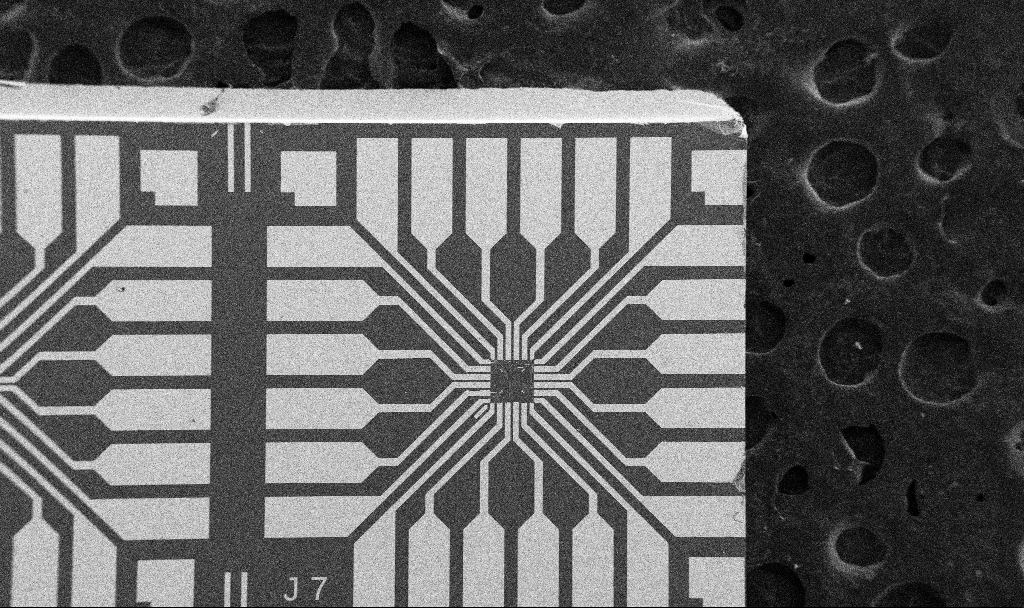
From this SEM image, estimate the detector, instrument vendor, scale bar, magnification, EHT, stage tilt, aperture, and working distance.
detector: SE2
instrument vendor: Zeiss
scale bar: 200000 nm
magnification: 0.1 K X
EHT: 5 kV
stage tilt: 0°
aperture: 30 µm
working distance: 10.7 mm